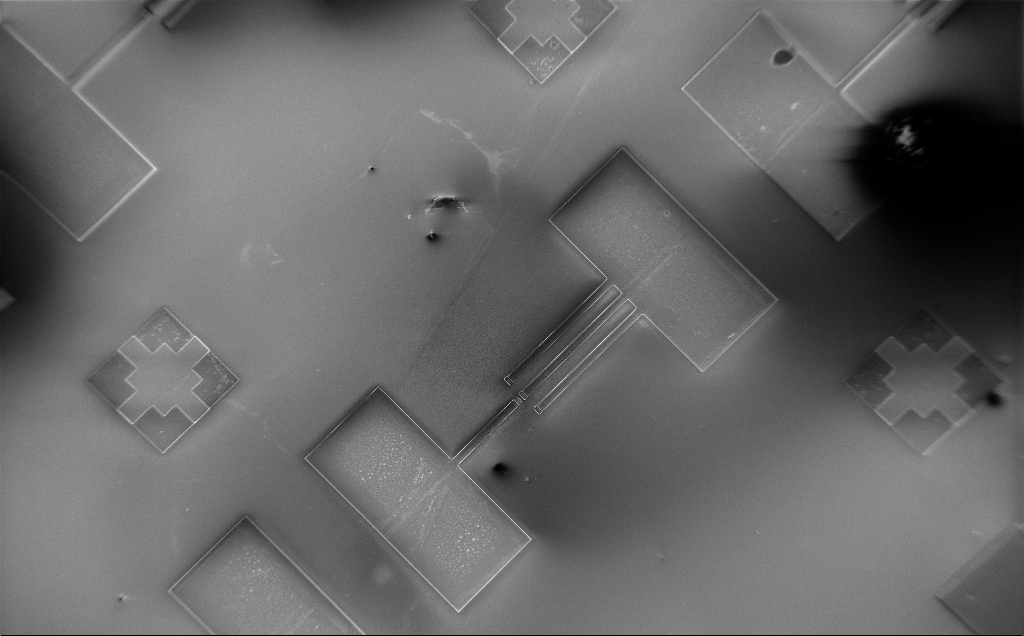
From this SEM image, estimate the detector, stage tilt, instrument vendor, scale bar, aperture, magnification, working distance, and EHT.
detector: InLens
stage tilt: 0°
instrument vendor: Zeiss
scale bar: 200000 nm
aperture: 30 µm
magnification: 0.19 K X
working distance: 10 mm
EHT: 2 kV